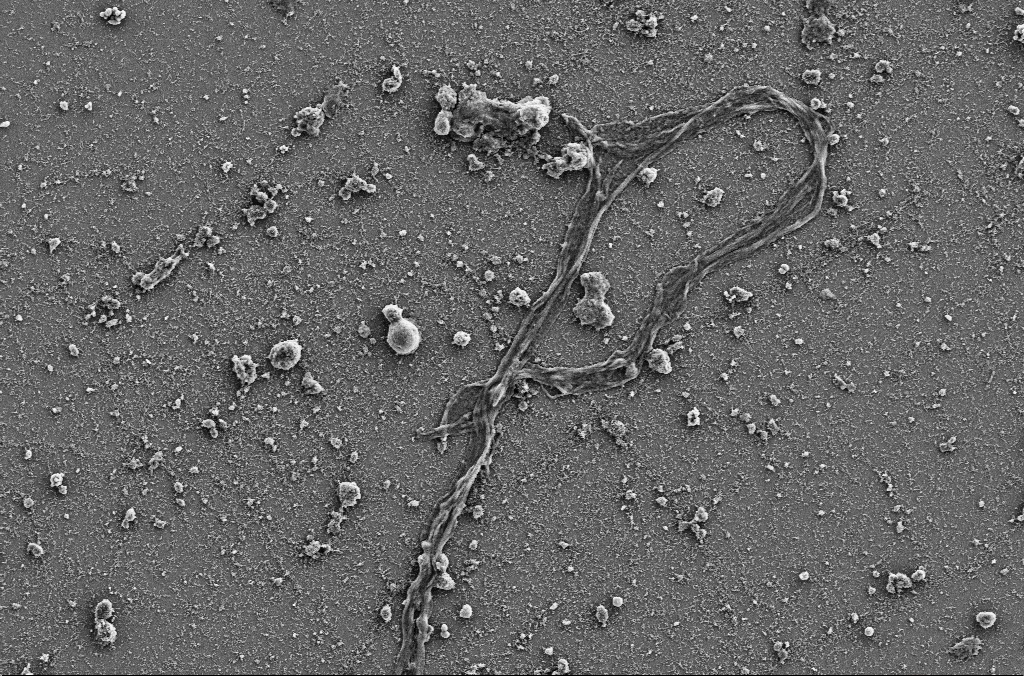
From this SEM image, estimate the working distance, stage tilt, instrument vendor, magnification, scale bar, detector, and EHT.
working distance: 4 mm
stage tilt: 0°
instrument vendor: Zeiss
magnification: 2.5 K X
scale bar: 10000 nm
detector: SE2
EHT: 5 kV